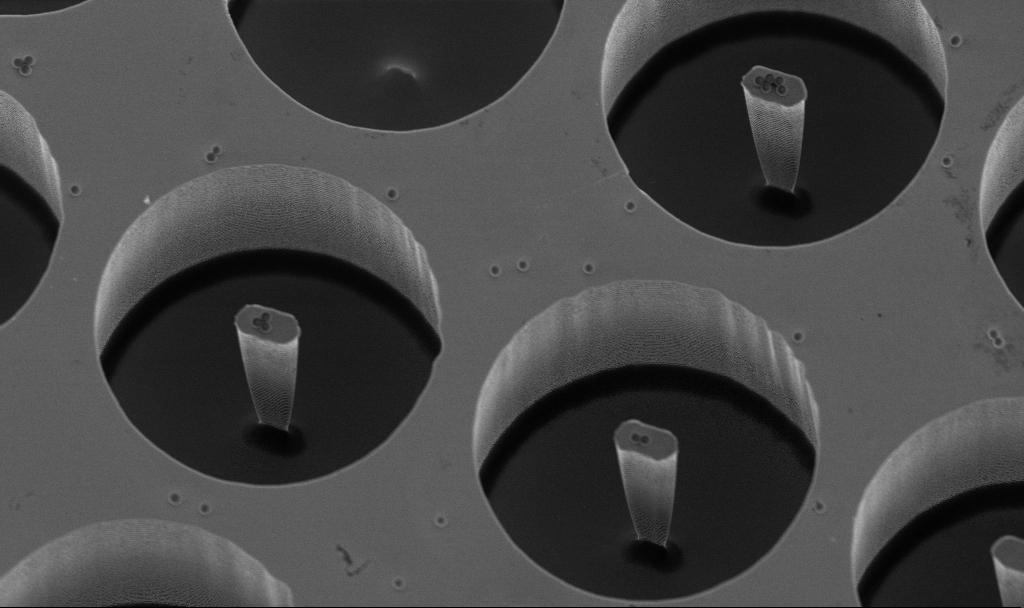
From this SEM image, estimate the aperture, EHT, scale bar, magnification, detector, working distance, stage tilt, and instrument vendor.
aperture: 30 µm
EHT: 3 kV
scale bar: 10000 nm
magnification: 6.4 K X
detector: InLens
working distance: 3.1 mm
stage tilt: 20°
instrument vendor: Zeiss